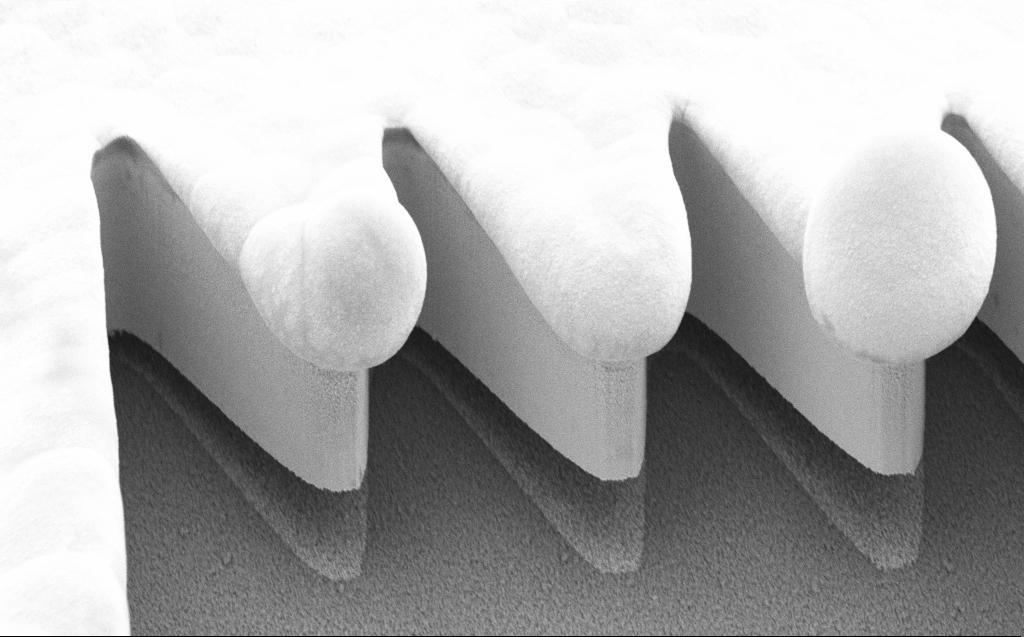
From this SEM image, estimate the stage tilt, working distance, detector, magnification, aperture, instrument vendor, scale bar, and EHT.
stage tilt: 45°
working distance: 9 mm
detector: SE2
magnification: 8.96 K X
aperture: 30 µm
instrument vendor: Zeiss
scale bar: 2000 nm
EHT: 5 kV